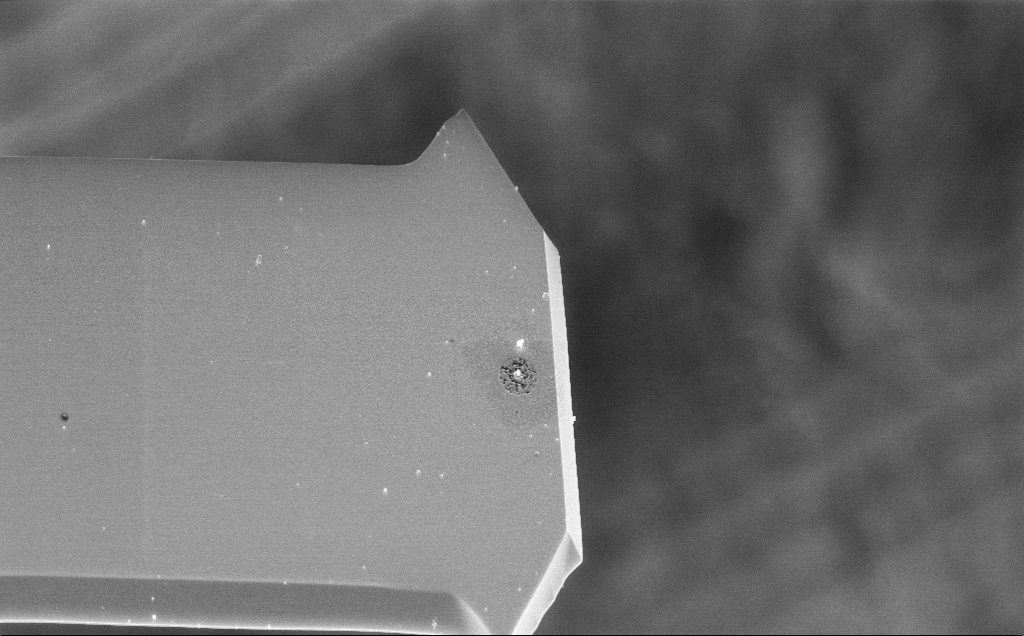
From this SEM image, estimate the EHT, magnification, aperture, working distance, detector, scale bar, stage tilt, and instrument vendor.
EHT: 10 kV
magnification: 4.63 K X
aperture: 30 µm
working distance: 3 mm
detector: InLens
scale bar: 10000 nm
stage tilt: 44.2°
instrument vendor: Zeiss